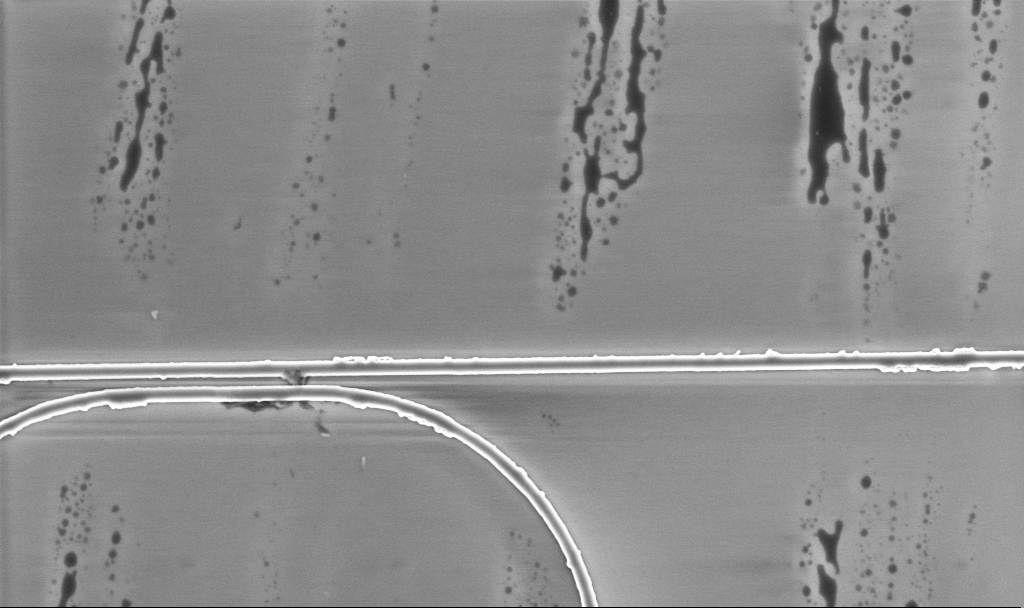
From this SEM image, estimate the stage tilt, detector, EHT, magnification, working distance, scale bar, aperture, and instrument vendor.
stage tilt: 0°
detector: InLens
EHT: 5 kV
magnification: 10.85 K X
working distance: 5.2 mm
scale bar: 2000 nm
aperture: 30 µm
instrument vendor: Zeiss